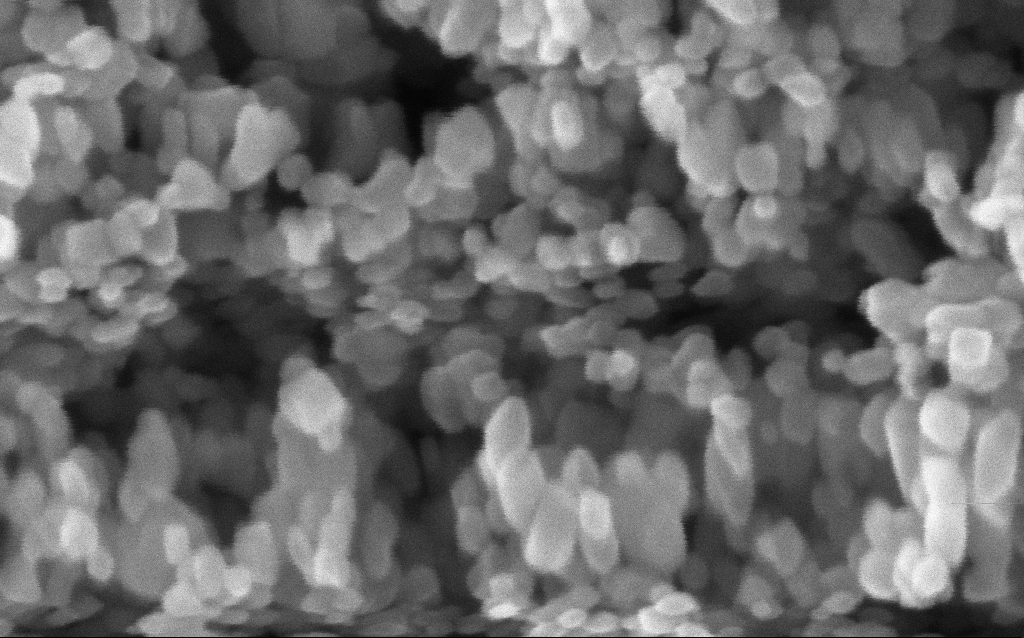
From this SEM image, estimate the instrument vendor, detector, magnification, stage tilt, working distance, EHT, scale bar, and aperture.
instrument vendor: Zeiss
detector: InLens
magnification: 600 K X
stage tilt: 0°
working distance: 2.9 mm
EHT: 5 kV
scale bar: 100 nm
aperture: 30 µm